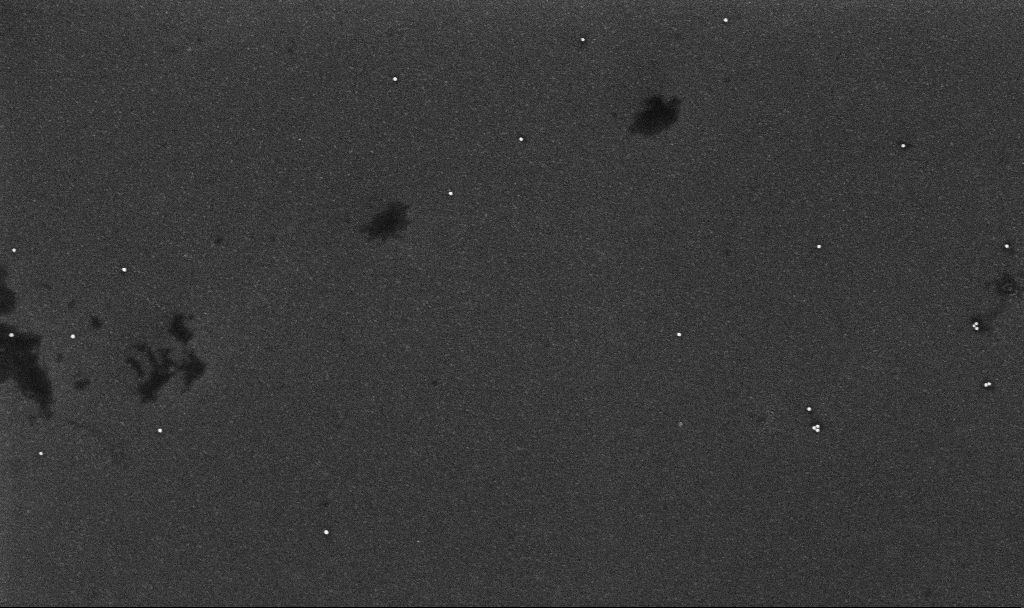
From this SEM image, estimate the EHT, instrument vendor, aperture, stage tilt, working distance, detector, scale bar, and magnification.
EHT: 10 kV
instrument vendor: Zeiss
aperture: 30 µm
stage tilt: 0°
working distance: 3.2 mm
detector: InLens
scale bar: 1000 nm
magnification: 53.52 K X